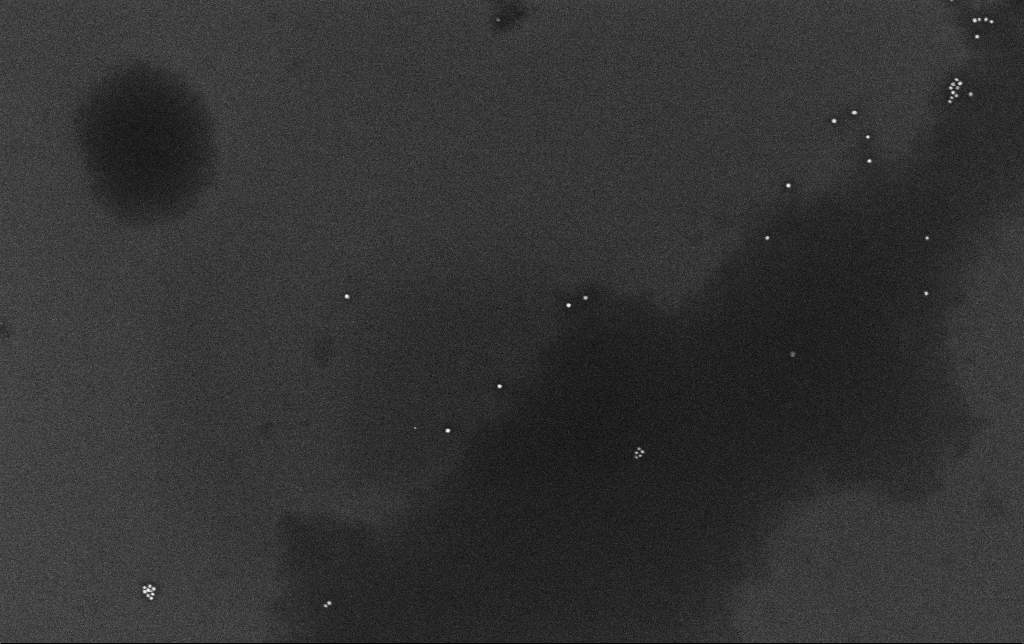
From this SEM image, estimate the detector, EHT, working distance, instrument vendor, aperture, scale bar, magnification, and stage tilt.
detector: InLens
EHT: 10 kV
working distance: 3.4 mm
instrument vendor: Zeiss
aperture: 30 µm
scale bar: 200 nm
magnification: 100 K X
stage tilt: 0°